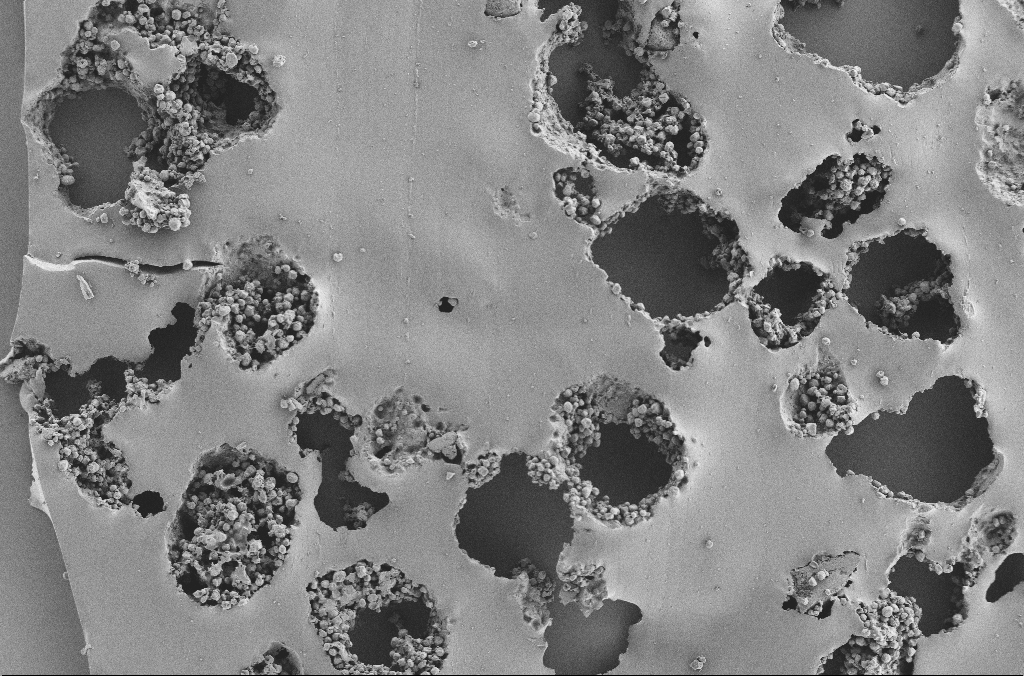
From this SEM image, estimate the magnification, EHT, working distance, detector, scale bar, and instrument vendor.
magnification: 0.25 K X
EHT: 2 kV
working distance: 3.7 mm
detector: SE2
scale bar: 100000 nm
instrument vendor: Zeiss